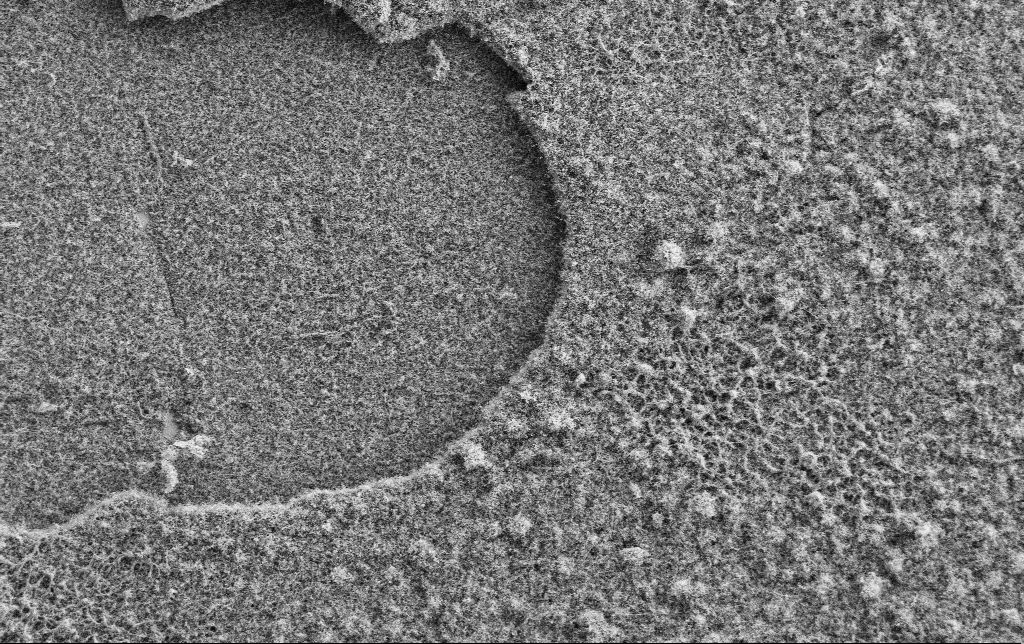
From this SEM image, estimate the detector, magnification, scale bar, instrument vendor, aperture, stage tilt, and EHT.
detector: SE2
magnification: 1 K X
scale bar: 20000 nm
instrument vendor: Zeiss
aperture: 30 µm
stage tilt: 0°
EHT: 2 kV